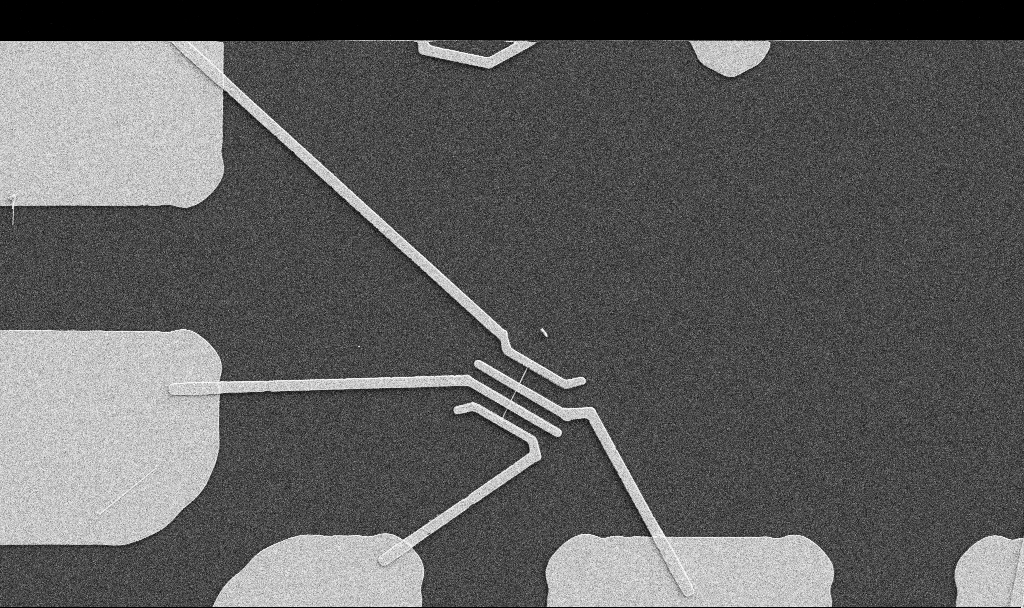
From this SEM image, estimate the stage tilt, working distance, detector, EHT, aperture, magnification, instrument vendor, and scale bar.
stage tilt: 0°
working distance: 10.7 mm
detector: SE2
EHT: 5 kV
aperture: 30 µm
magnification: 5 K X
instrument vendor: Zeiss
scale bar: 10000 nm